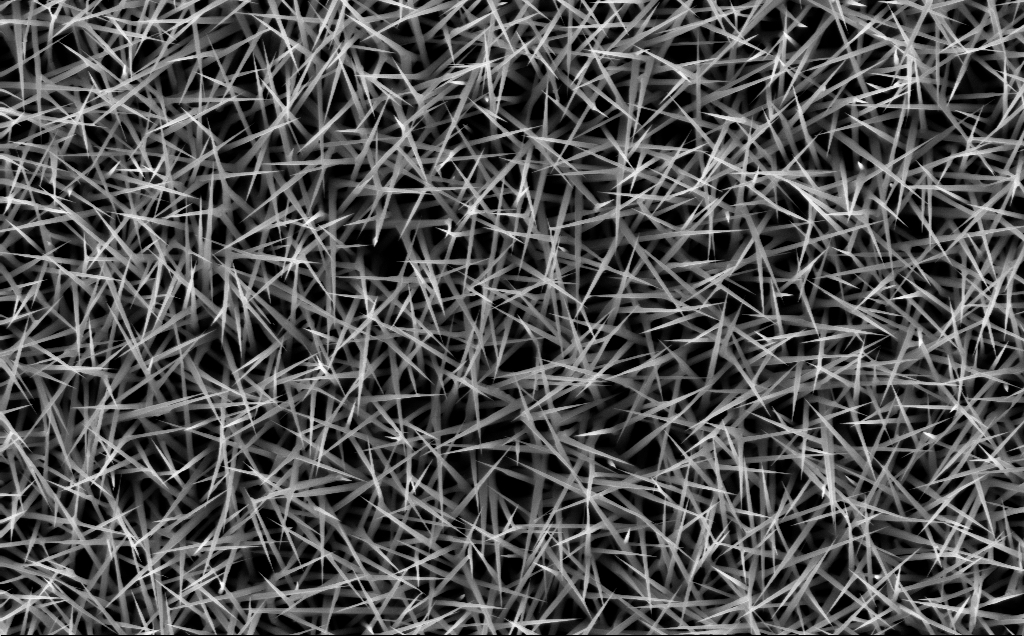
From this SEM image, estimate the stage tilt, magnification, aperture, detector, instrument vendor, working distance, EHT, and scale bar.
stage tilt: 0°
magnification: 20 K X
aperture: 30 µm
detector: InLens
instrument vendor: Zeiss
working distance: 7 mm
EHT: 10 kV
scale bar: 1000 nm